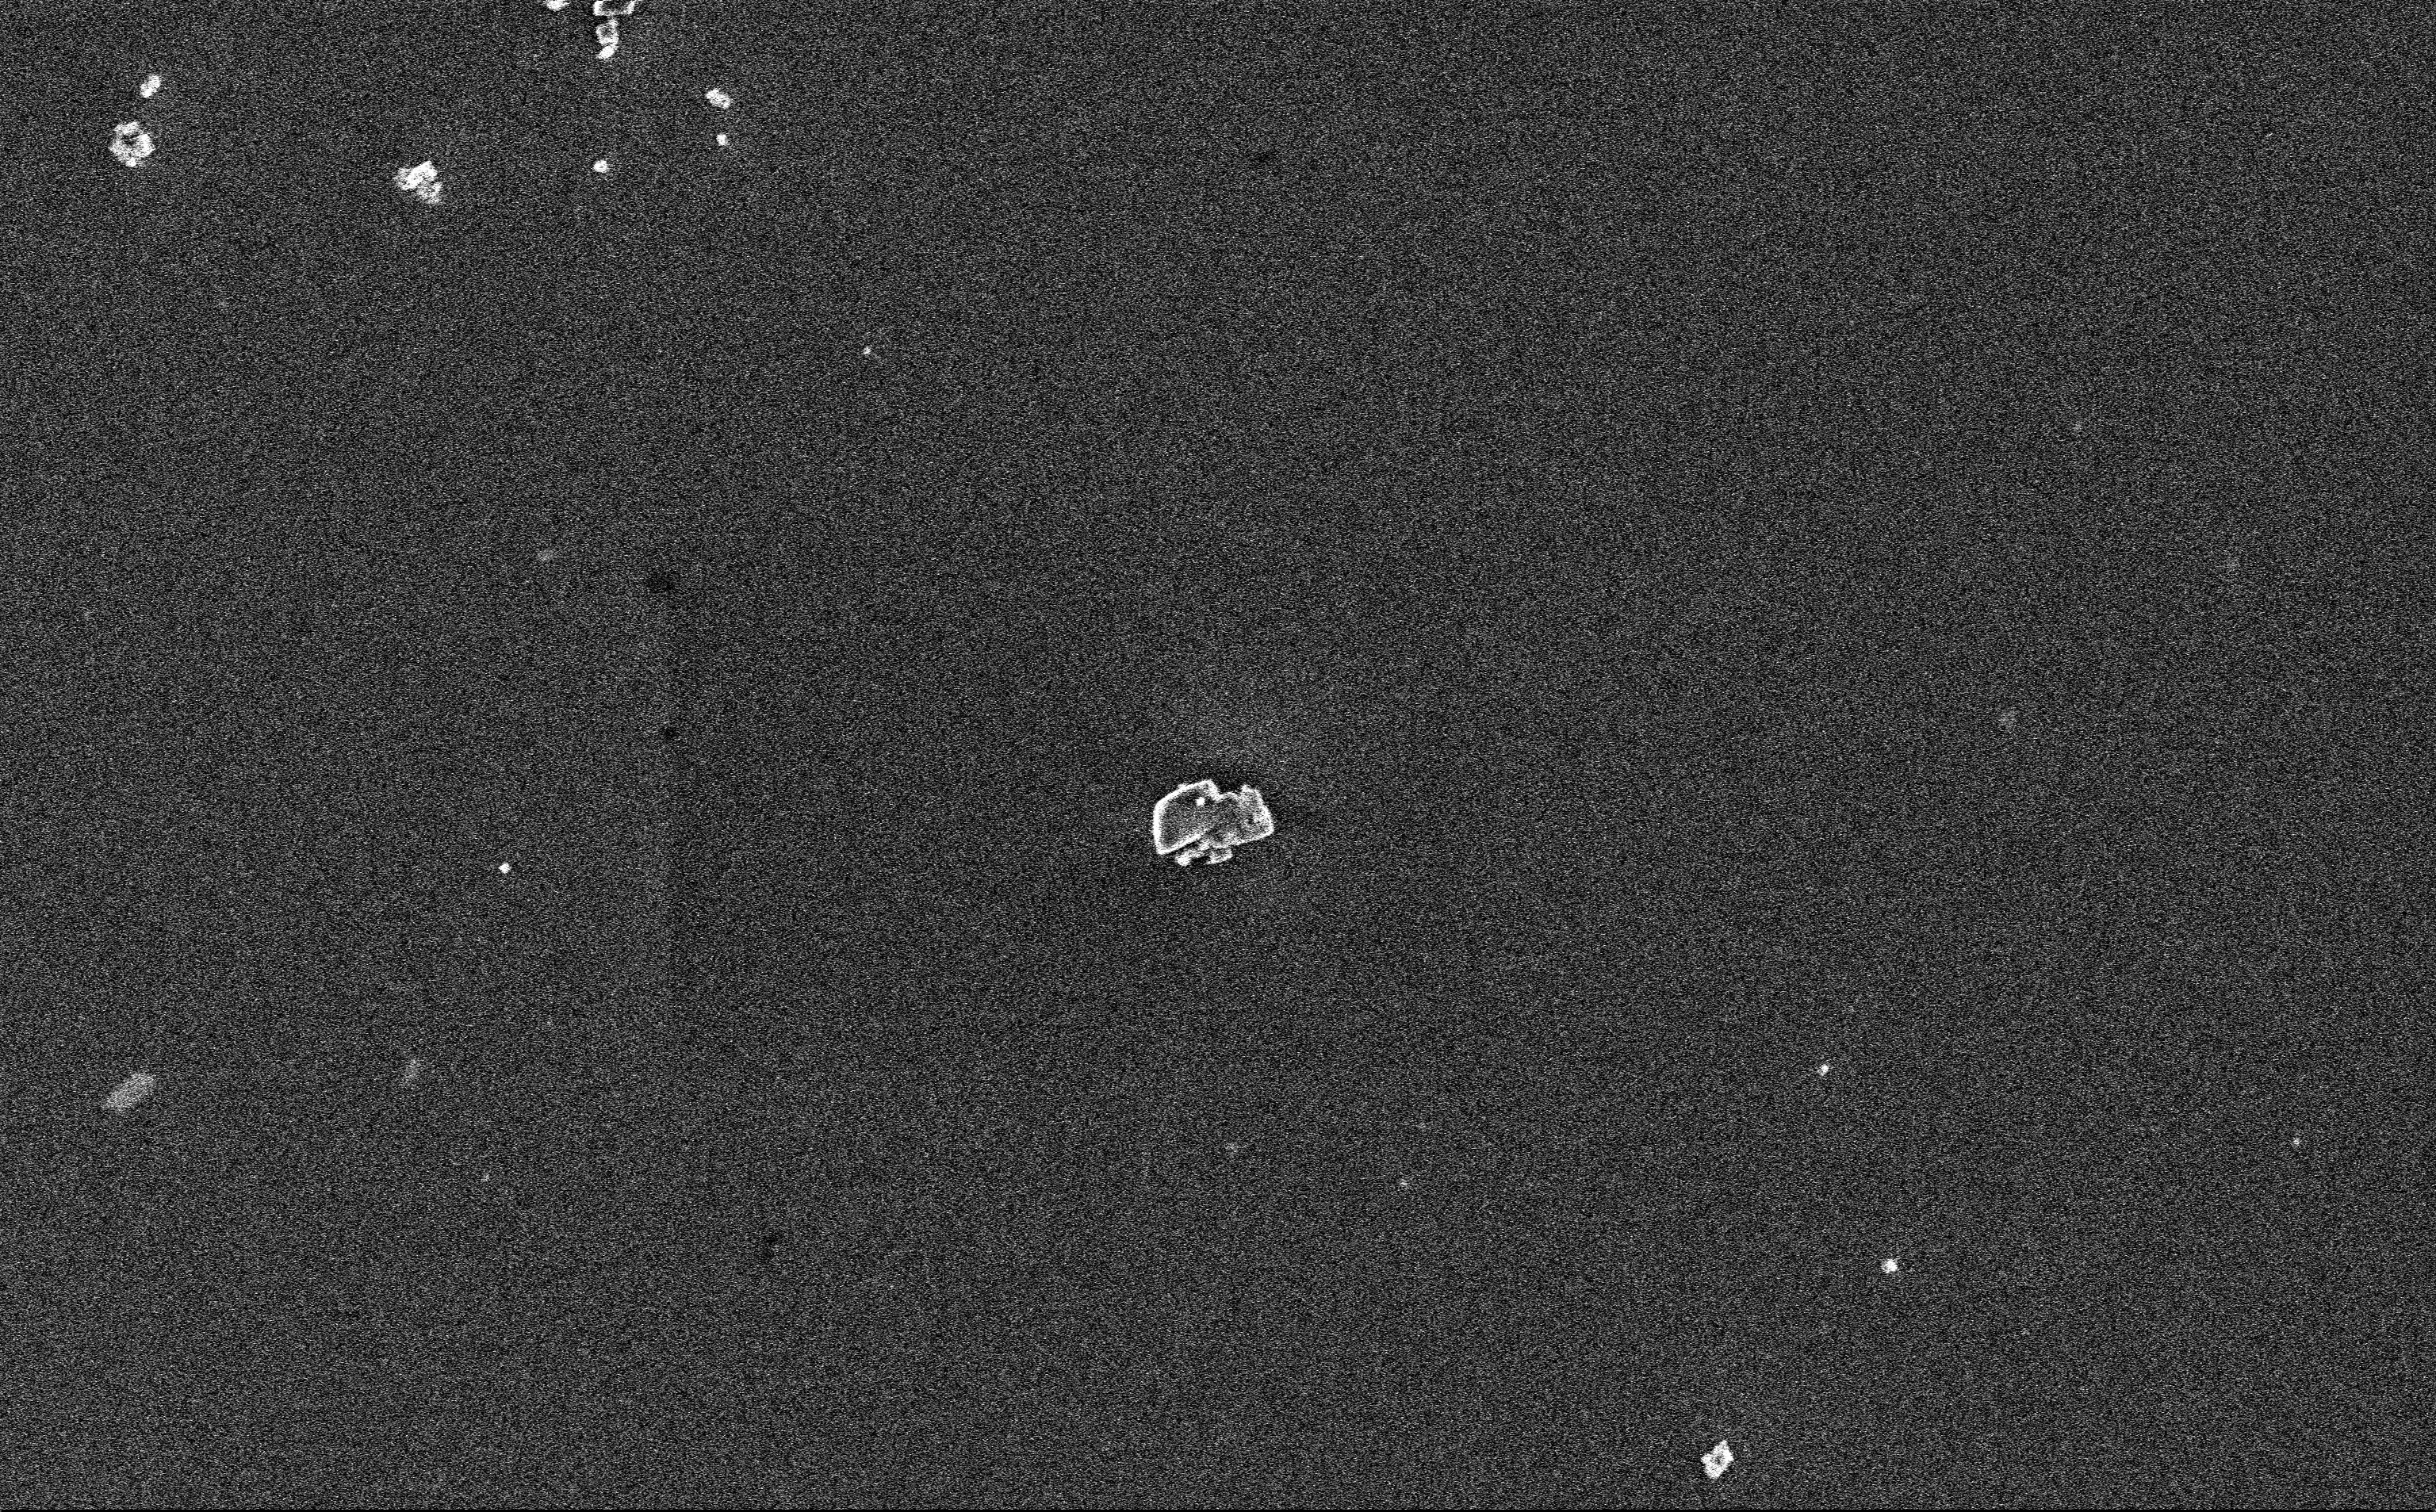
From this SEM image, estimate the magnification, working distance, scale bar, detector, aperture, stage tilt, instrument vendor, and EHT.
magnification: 12.85 K X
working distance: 3 mm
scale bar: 2000 nm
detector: InLens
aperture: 30 µm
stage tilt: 0°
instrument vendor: Zeiss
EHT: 3 kV